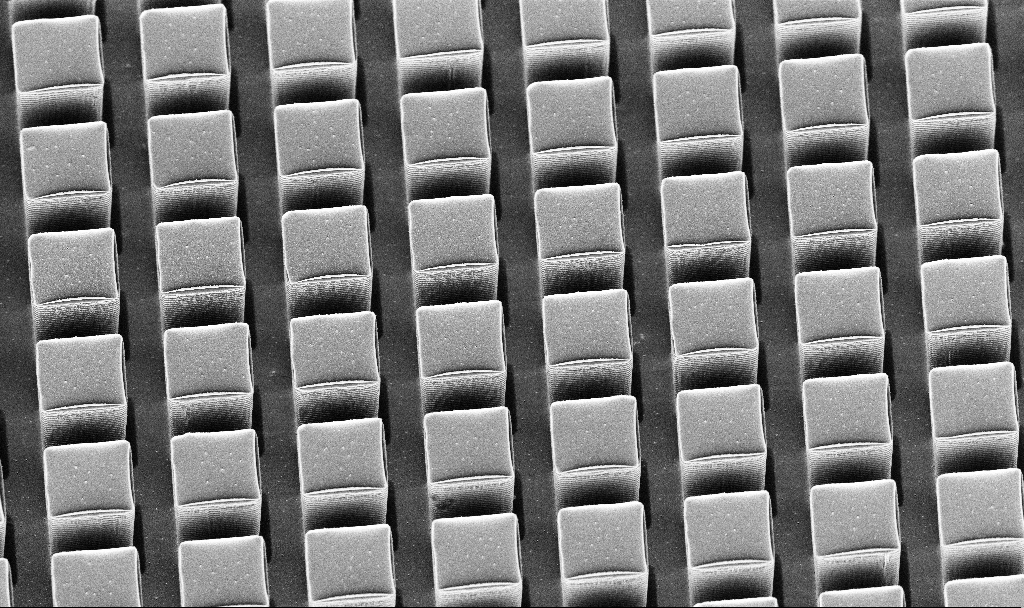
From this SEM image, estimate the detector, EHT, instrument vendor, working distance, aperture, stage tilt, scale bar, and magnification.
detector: InLens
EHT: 5 kV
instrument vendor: Zeiss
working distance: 9.4 mm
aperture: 30 µm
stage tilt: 30°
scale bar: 10000 nm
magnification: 3.88 K X